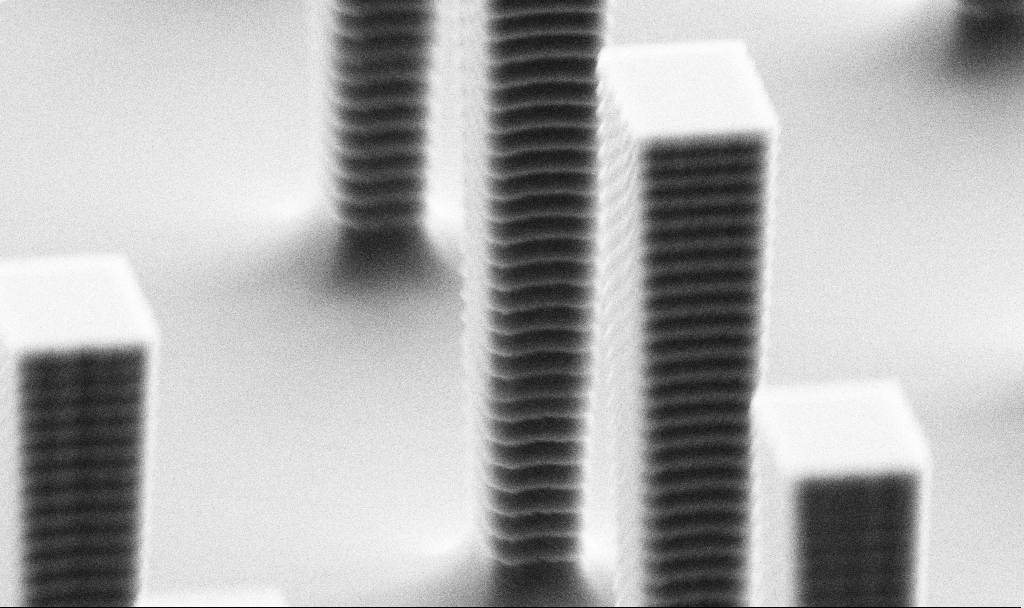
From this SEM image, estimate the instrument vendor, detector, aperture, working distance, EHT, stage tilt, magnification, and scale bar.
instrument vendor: Zeiss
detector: SE2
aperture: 30 µm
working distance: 6.1 mm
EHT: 5 kV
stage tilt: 70°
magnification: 28.62 K X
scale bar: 2000 nm